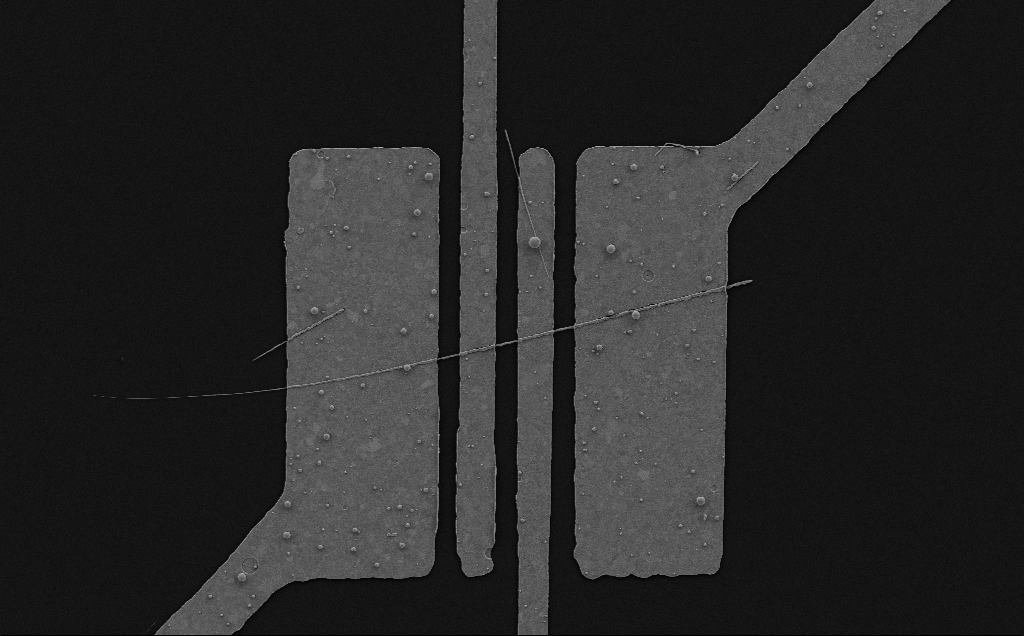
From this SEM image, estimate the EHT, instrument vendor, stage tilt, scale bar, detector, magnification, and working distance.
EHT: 5 kV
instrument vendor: Zeiss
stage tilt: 0°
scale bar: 10000 nm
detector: SE2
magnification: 5.36 K X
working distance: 6 mm